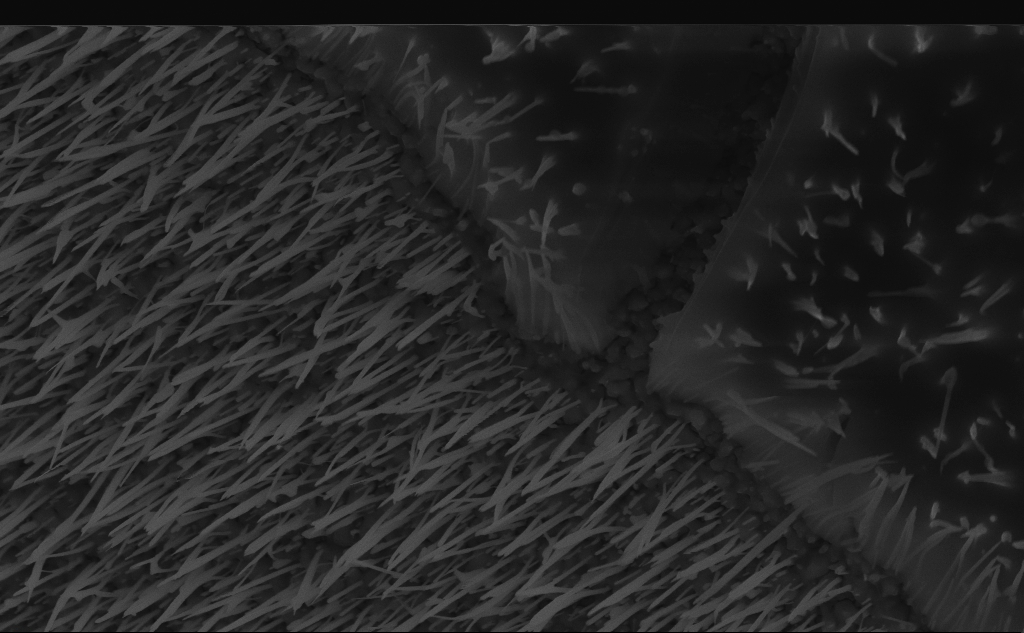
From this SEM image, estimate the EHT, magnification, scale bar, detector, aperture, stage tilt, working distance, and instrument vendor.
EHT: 10 kV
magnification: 35.6 K X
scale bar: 2000 nm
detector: InLens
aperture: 30 µm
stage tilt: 45°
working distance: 6 mm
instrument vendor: Zeiss